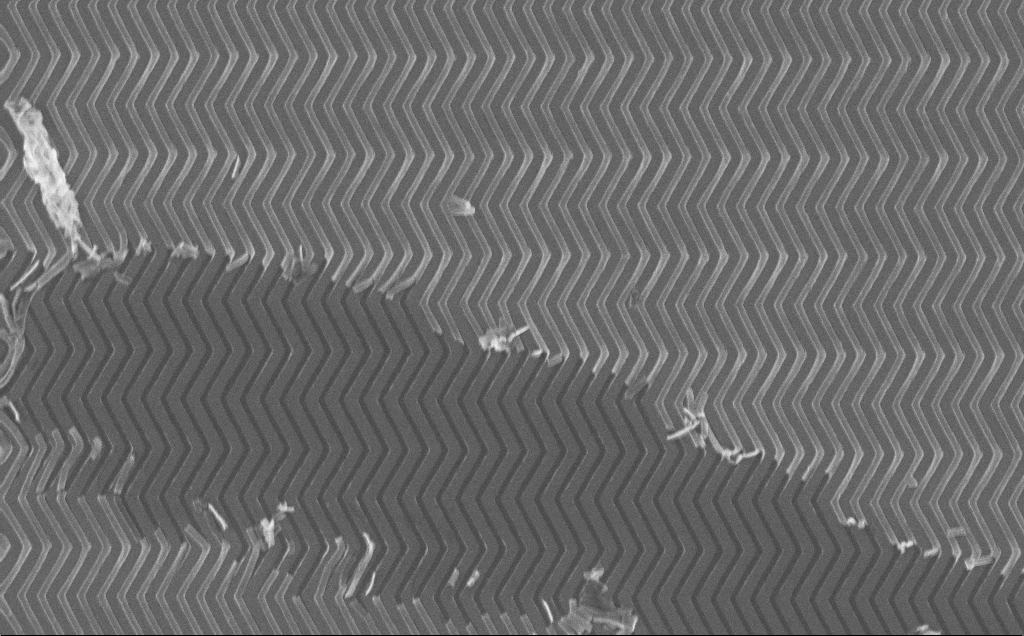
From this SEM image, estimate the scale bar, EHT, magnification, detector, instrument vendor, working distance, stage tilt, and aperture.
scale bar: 2000 nm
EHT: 10 kV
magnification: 18.2 K X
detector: InLens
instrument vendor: Zeiss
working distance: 7 mm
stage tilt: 0°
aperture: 30 µm